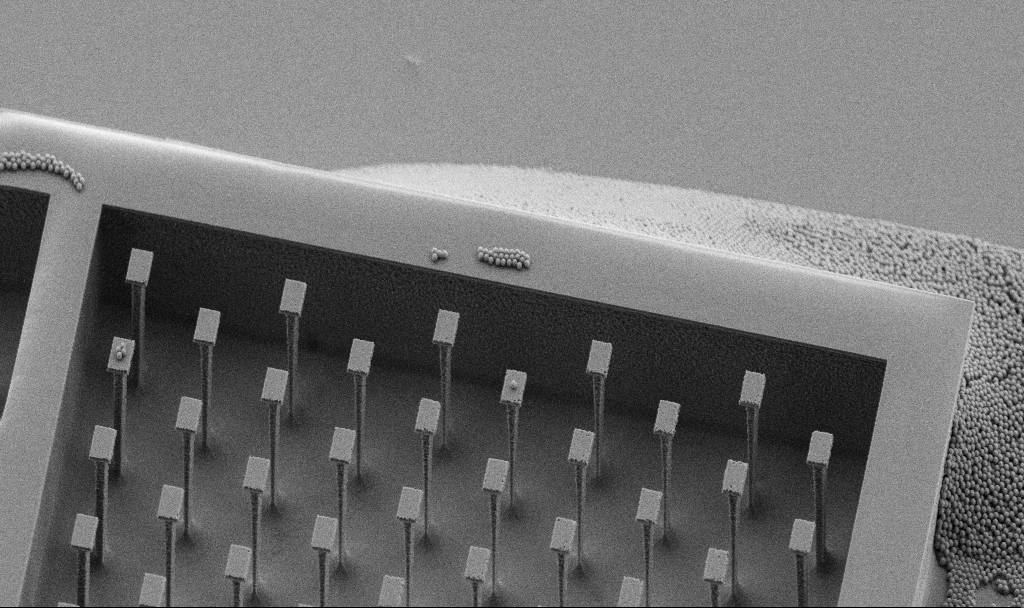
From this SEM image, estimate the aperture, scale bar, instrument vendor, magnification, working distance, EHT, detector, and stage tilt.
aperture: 30 µm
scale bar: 10000 nm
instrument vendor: Zeiss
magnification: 2.76 K X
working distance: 5.9 mm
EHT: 5 kV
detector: SE2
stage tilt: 45°